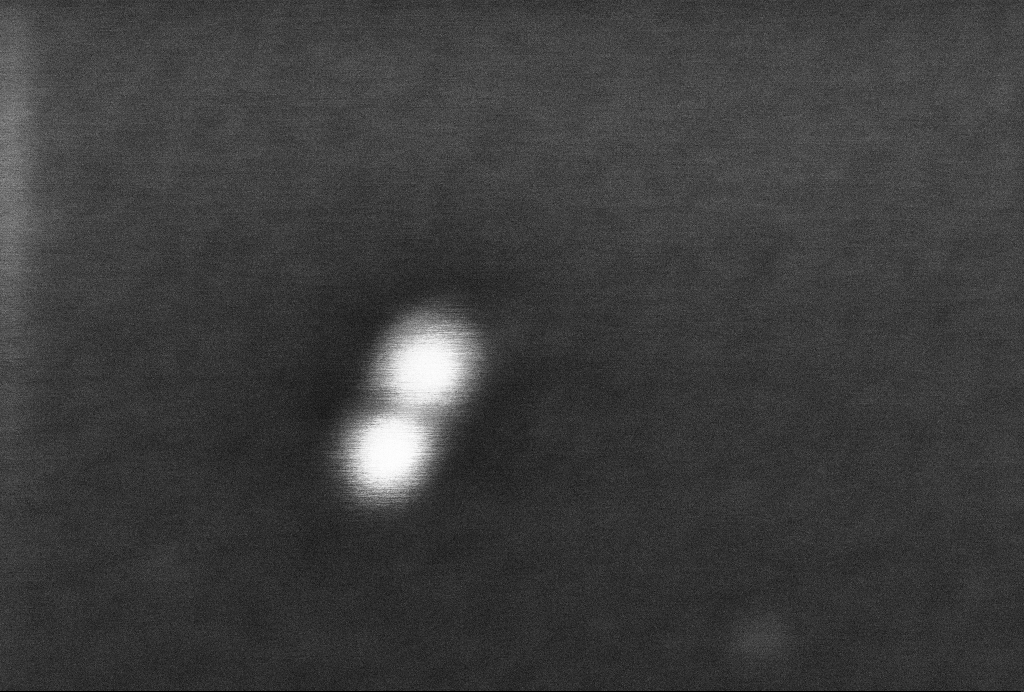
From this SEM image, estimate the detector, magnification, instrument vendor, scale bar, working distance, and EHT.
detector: InLens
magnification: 1212.12 K X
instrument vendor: Zeiss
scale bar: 20 nm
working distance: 3.3 mm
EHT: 2 kV